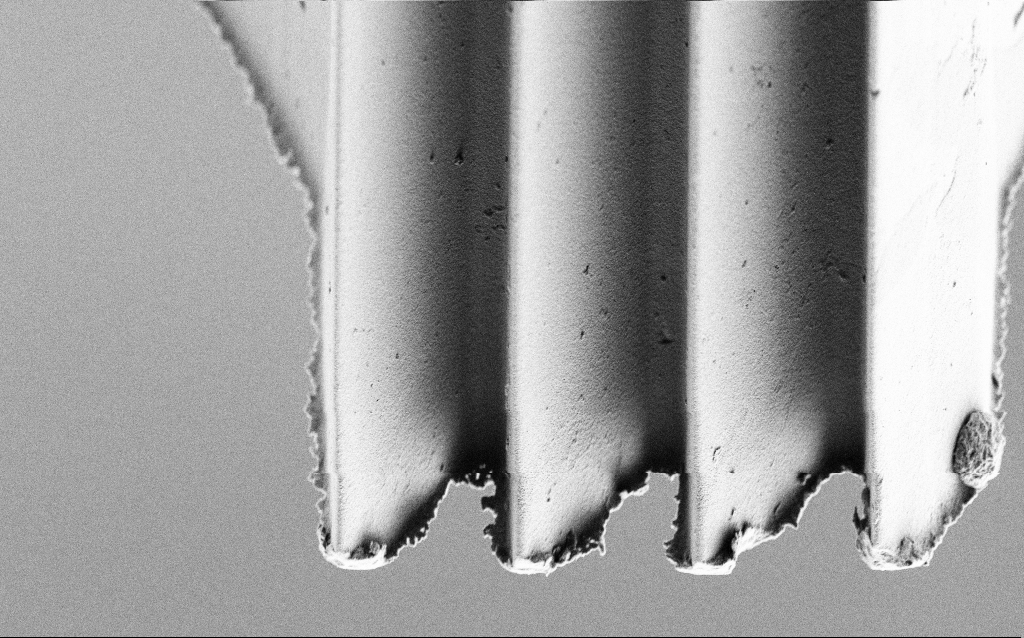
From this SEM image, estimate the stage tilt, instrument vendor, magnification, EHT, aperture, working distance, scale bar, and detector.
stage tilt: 45°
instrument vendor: Zeiss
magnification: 5.84 K X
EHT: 3 kV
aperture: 30 µm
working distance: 4 mm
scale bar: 10000 nm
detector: SE2